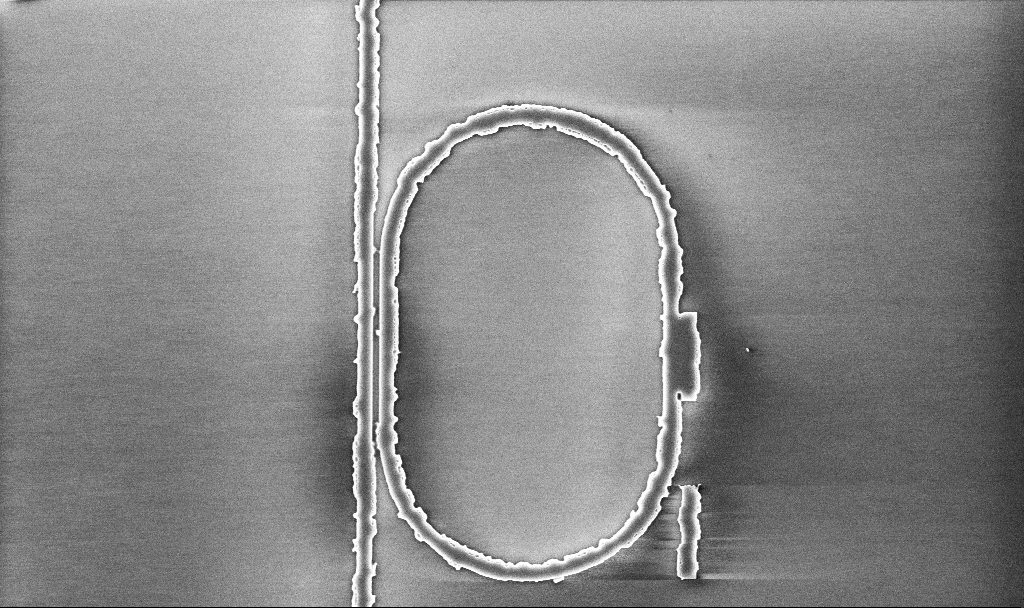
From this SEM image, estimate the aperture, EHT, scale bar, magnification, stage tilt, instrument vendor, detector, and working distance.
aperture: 30 µm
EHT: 5 kV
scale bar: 2000 nm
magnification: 11.04 K X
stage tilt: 0°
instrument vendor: Zeiss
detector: InLens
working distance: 10.1 mm